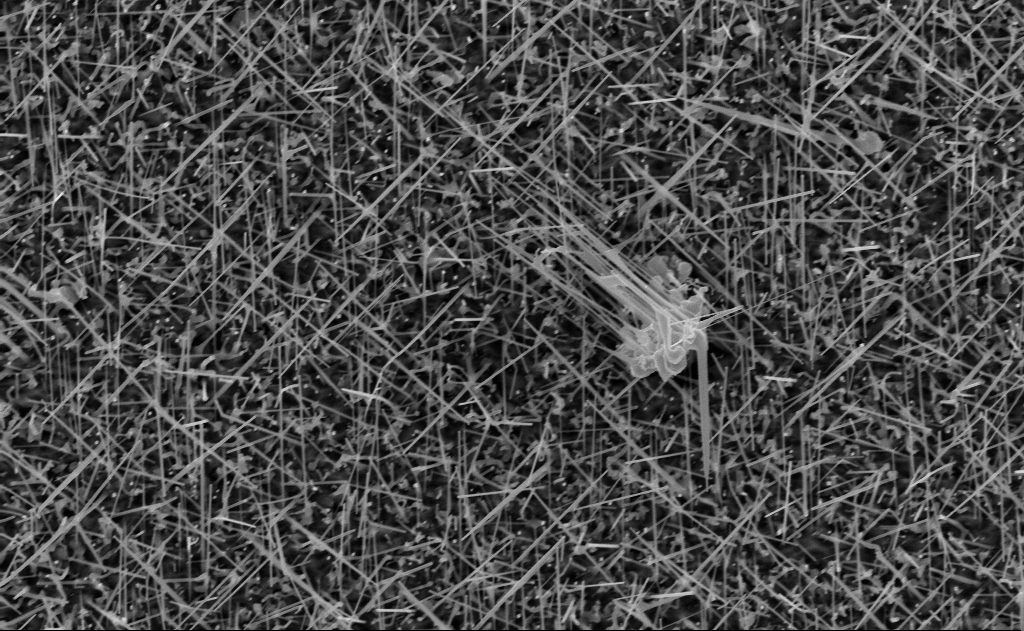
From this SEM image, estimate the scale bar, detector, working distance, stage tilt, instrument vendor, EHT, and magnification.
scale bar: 2000 nm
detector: InLens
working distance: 15 mm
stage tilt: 0°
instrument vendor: Zeiss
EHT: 10 kV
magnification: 20 K X